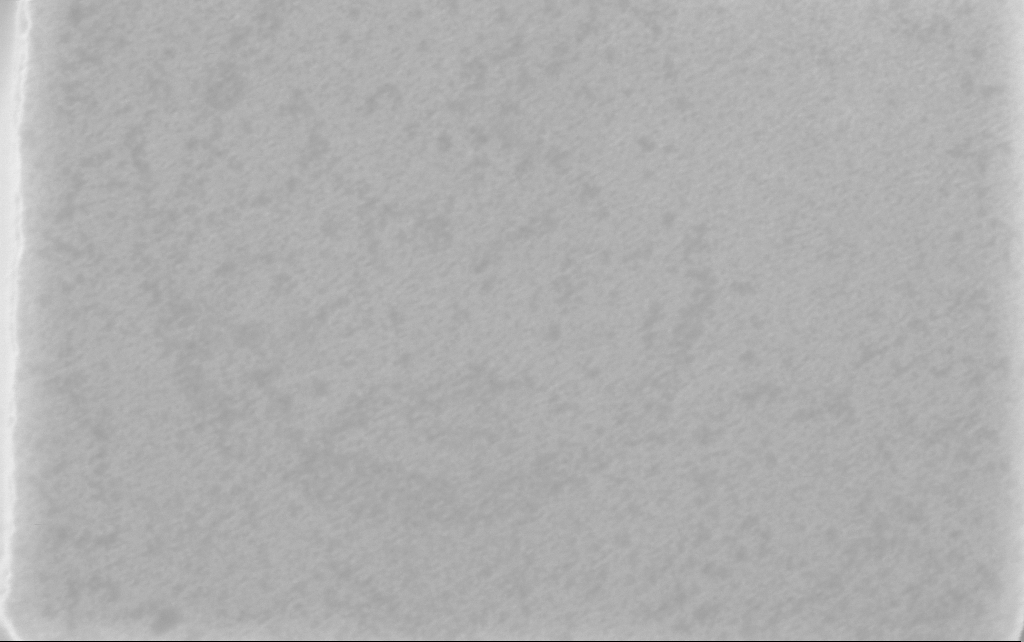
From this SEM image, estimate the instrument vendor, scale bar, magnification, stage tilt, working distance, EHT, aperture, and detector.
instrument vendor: Zeiss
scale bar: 200 nm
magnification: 124.43 K X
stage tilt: -0°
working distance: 2.5 mm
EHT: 3 kV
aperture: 30 µm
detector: InLens